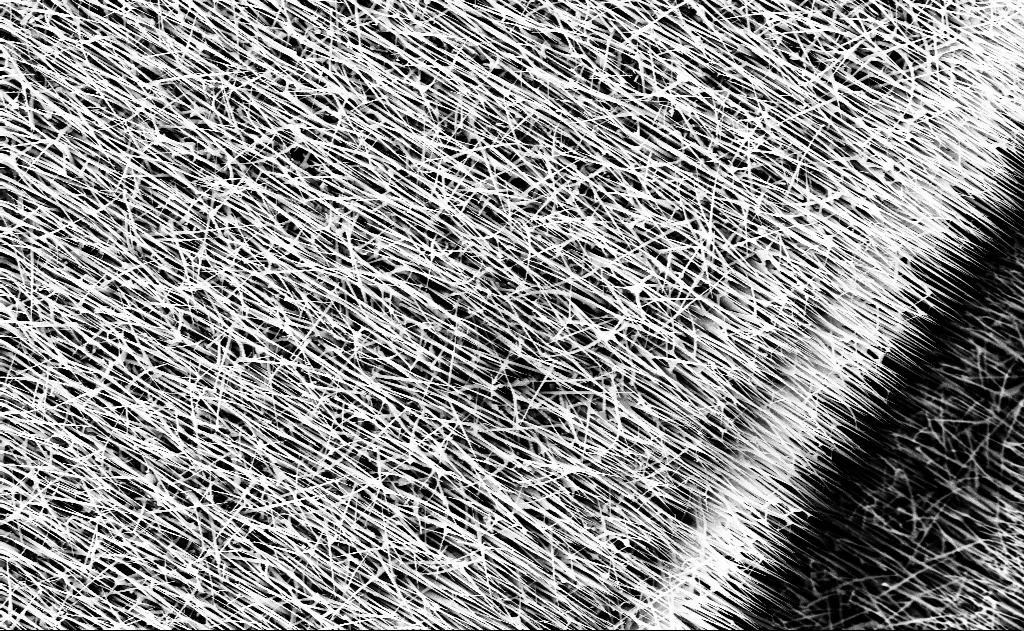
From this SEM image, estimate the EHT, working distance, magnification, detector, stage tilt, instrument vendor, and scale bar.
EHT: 10 kV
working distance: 13 mm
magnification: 10 K X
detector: InLens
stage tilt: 0°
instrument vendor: Zeiss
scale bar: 2000 nm